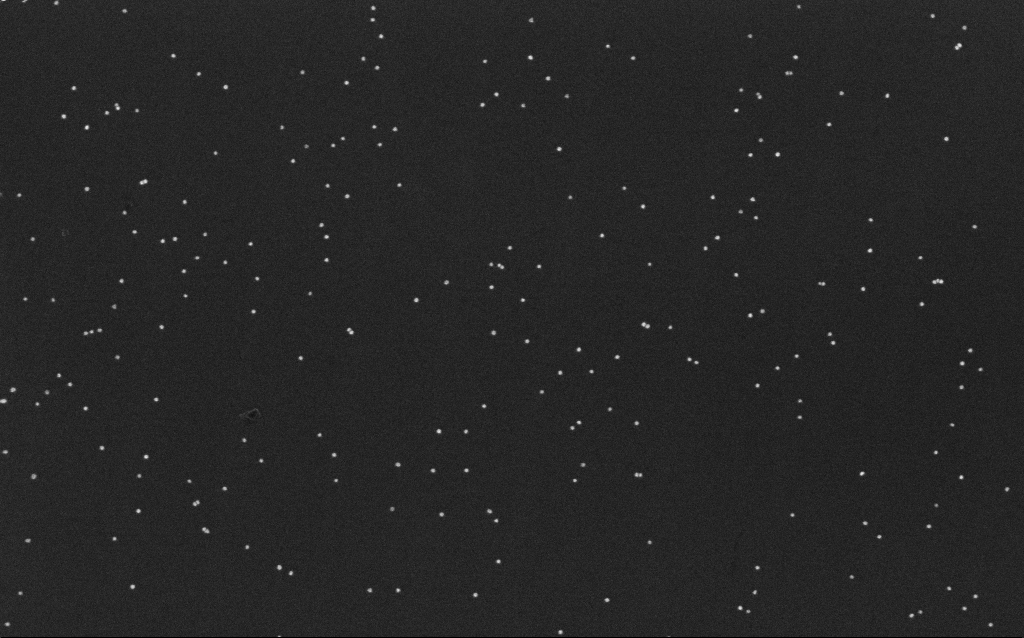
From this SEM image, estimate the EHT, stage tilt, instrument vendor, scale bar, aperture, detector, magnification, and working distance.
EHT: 10 kV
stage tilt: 0°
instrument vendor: Zeiss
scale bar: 200 nm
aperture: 30 µm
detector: InLens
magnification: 100 K X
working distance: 6.5 mm